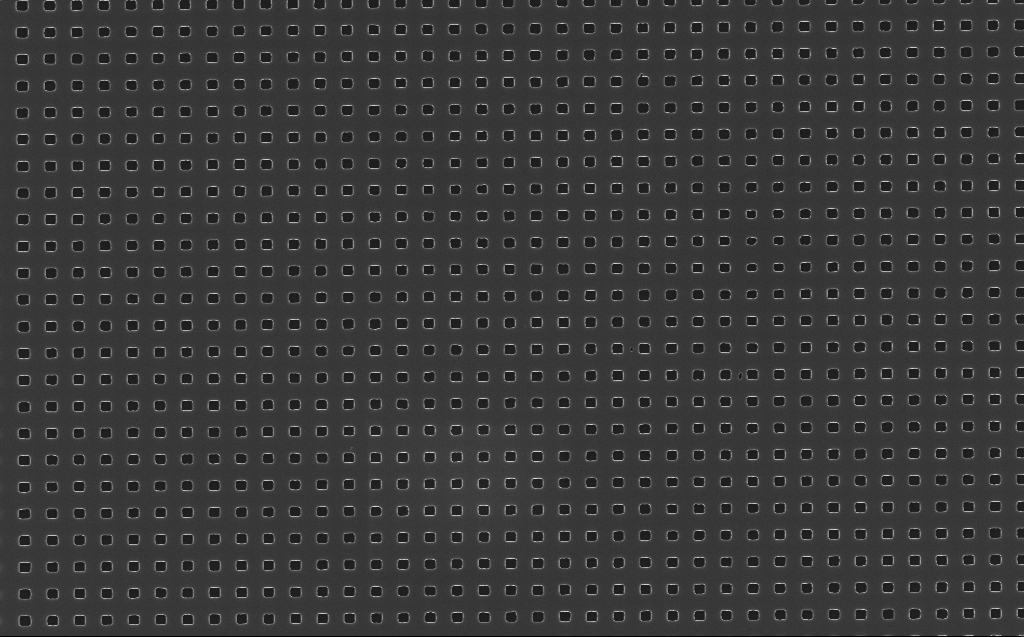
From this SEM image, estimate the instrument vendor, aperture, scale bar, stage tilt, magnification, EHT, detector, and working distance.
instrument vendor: Zeiss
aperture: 30 µm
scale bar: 1000 nm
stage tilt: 0°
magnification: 20 K X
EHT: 10 kV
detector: InLens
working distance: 6 mm